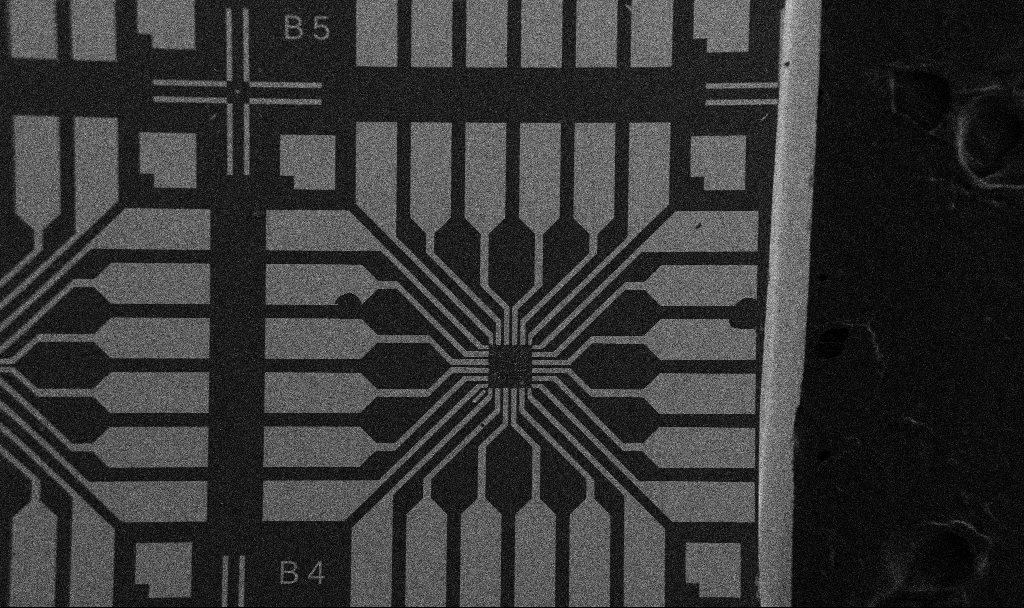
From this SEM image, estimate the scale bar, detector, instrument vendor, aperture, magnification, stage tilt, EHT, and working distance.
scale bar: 200000 nm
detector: SE2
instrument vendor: Zeiss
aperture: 30 µm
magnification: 0.1 K X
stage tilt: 0°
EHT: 5 kV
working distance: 10.7 mm